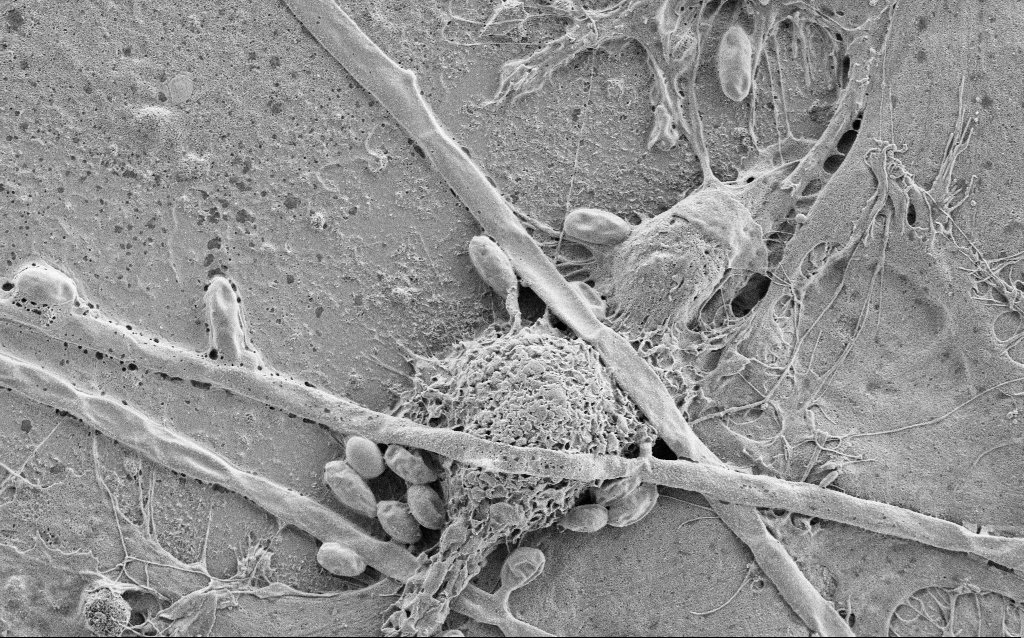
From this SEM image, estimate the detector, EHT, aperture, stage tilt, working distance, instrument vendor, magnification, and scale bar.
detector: SE2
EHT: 2 kV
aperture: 30 µm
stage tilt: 0°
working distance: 6.8 mm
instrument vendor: Zeiss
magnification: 5 K X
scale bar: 10000 nm